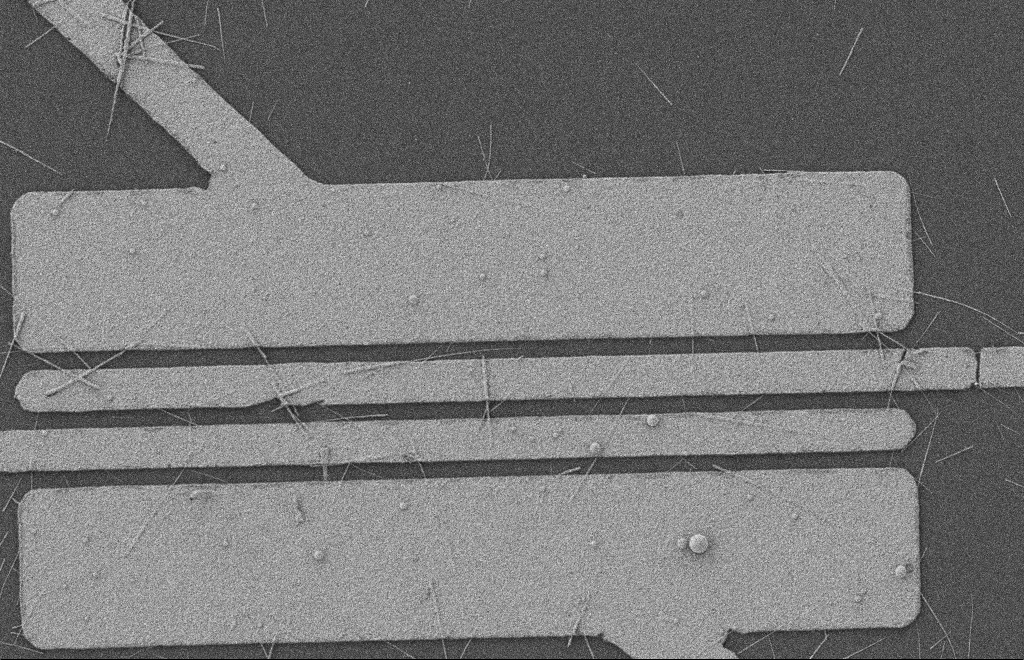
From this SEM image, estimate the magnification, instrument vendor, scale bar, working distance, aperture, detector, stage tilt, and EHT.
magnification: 5.4 K X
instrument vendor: Zeiss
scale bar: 2000 nm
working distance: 8 mm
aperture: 20 µm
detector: SE2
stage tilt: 0°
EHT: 2 kV